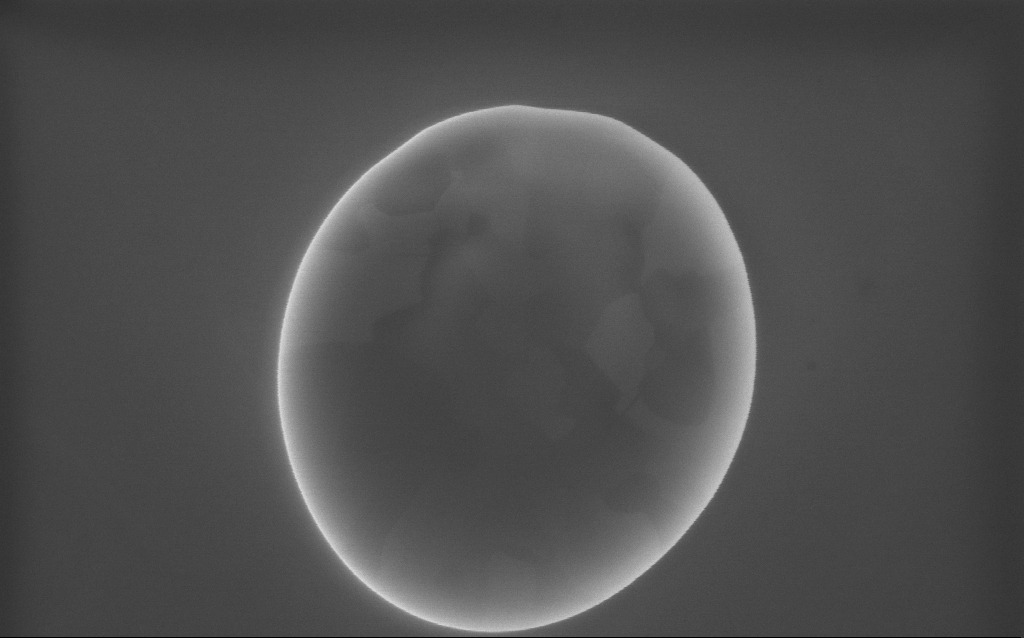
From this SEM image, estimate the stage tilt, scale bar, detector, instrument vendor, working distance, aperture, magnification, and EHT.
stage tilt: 0°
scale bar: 200 nm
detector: InLens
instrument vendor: Zeiss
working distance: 2 mm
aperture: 30 µm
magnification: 97 K X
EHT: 10 kV